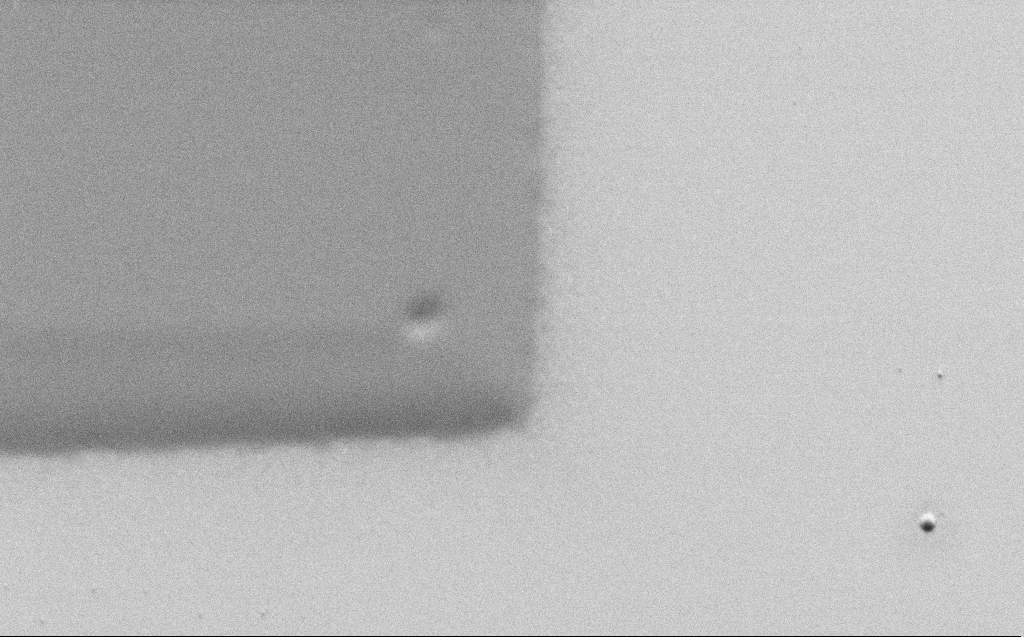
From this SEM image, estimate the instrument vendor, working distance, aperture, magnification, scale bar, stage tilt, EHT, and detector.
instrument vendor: Zeiss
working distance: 5 mm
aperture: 30 µm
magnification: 13.42 K X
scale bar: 2000 nm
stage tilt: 45°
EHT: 1 kV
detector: SE2